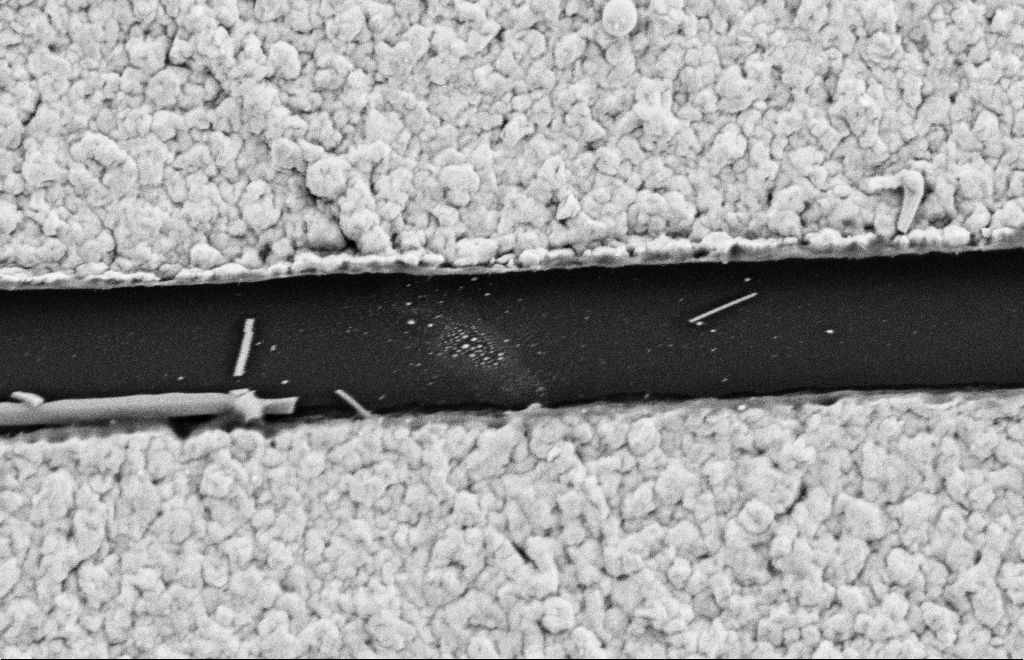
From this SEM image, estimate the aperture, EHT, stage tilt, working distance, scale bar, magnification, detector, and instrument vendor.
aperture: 20 µm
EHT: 2 kV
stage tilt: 0°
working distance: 9 mm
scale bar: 200 nm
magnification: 72.75 K X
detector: SE2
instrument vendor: Zeiss